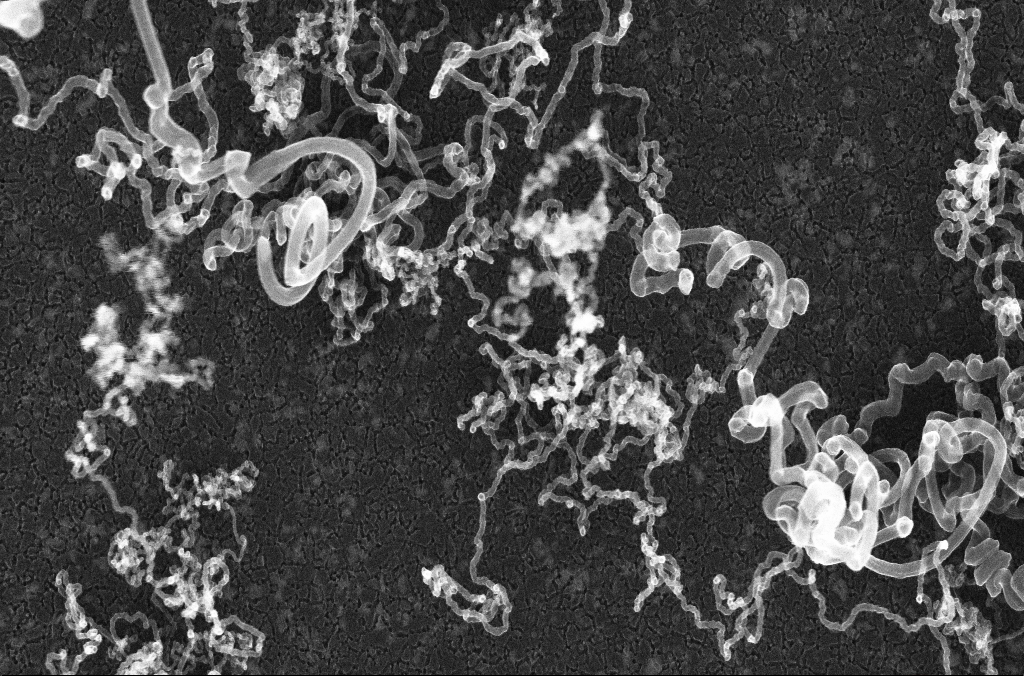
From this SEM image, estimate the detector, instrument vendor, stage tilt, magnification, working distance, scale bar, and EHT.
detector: InLens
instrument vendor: Zeiss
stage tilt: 0°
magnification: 50 K X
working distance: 4.3 mm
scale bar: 1000 nm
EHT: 20 kV